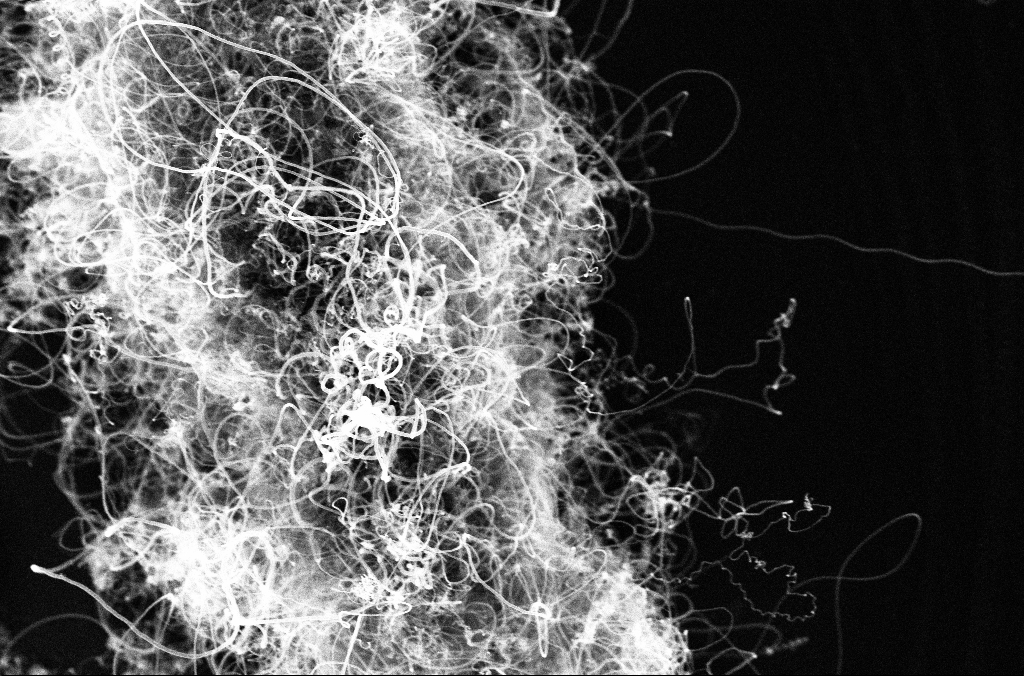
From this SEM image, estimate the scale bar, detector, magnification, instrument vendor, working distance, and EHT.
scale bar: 1000 nm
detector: InLens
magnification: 25 K X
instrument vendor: Zeiss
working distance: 3.3 mm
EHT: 10 kV